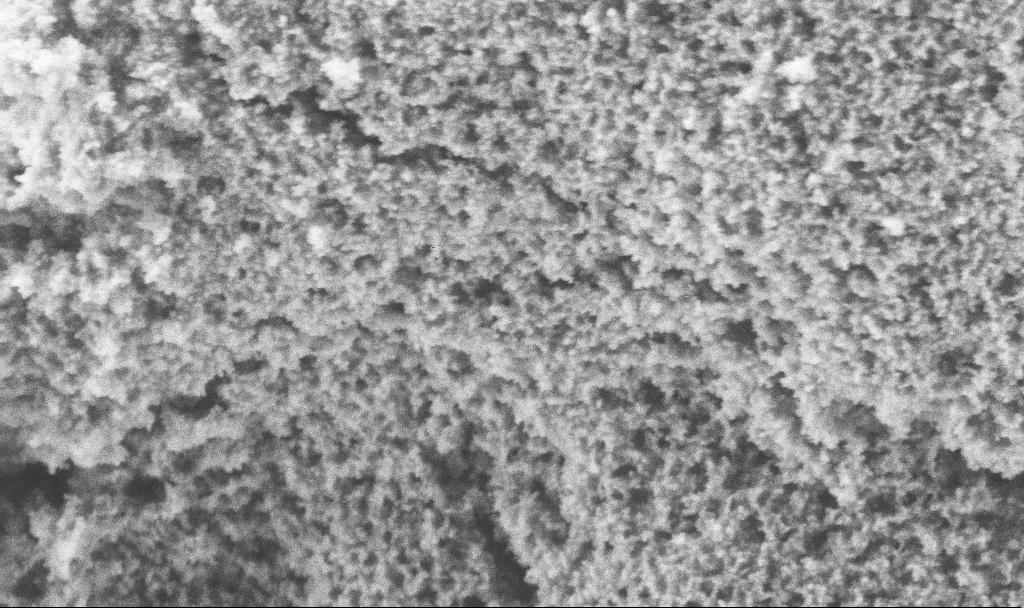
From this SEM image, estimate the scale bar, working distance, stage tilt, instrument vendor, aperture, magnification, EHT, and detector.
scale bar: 1000 nm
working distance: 2.7 mm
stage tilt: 0°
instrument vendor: Zeiss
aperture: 30 µm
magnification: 55.95 K X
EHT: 3 kV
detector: SE2